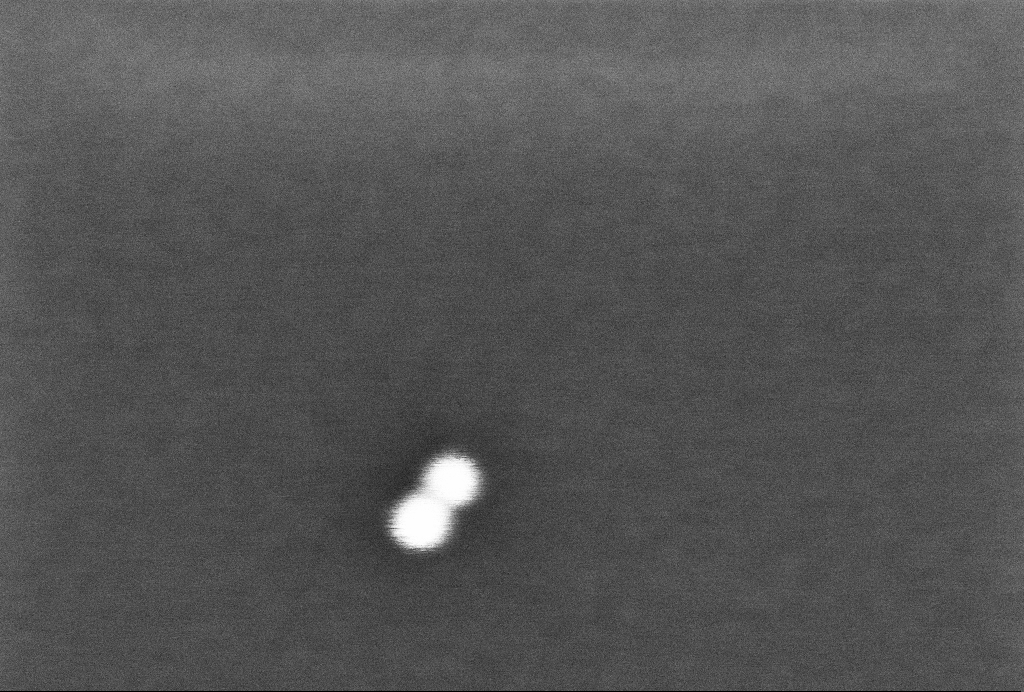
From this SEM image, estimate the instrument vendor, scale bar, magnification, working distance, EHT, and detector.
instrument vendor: Zeiss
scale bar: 20 nm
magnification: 650.78 K X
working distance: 3.3 mm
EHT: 2 kV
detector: InLens